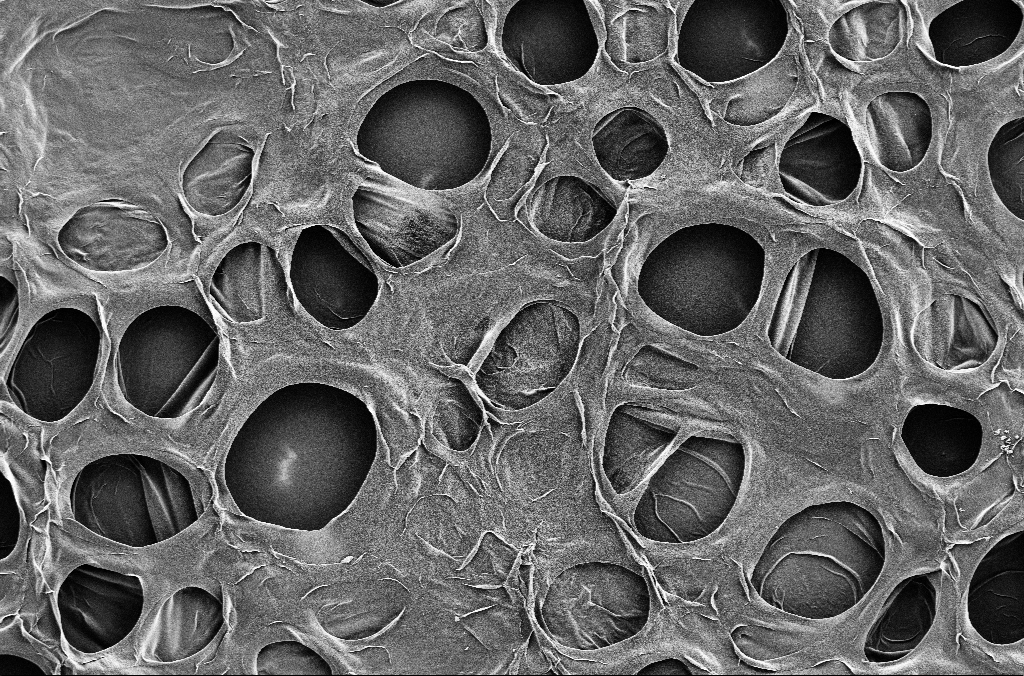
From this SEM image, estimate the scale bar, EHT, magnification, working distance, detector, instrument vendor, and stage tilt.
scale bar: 20000 nm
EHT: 1.8 kV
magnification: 1 K X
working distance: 5.7 mm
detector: SE2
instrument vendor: Zeiss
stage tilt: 0°